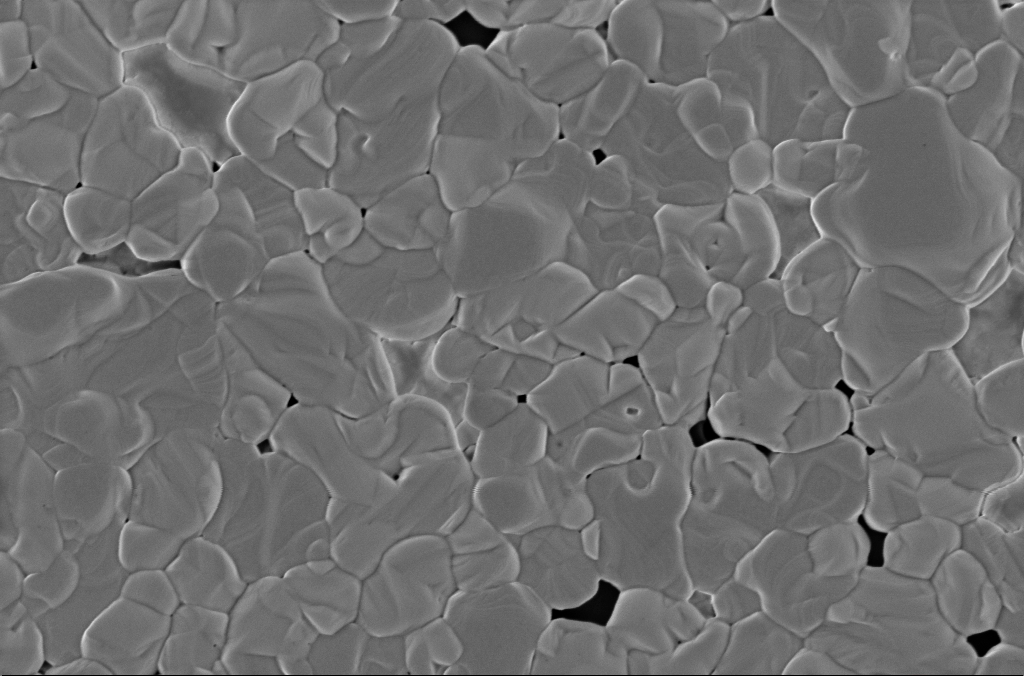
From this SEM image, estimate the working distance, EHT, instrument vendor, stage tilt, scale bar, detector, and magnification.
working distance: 3 mm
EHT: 2 kV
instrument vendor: Zeiss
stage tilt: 0°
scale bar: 1000 nm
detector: InLens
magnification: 50 K X